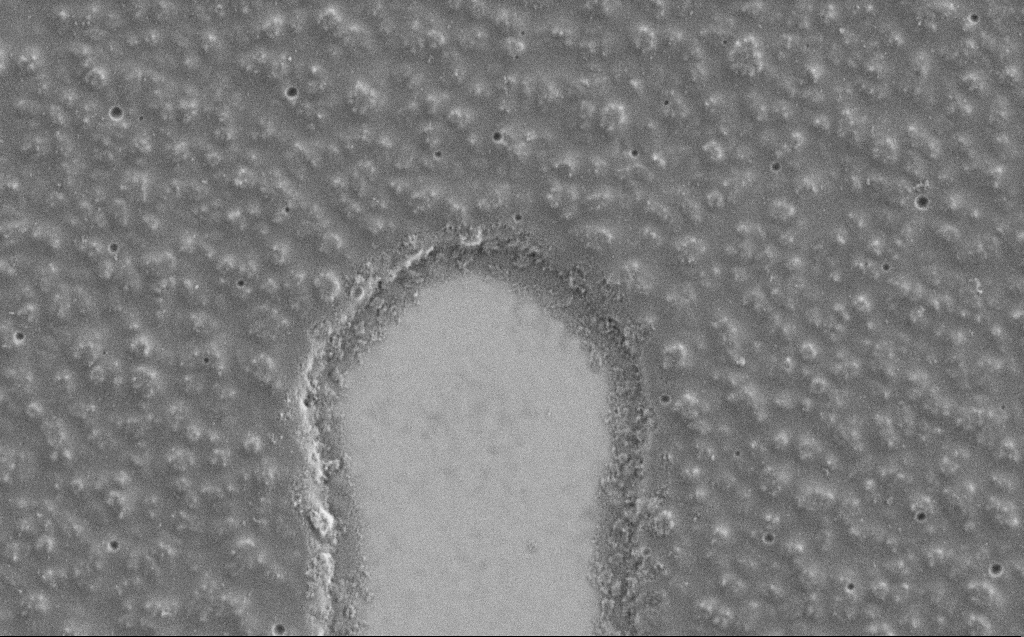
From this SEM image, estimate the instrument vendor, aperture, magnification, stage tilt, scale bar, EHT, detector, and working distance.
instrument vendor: Zeiss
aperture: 30 µm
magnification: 26.28 K X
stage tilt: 0°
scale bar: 2000 nm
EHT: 1.2 kV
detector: SE2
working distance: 4 mm